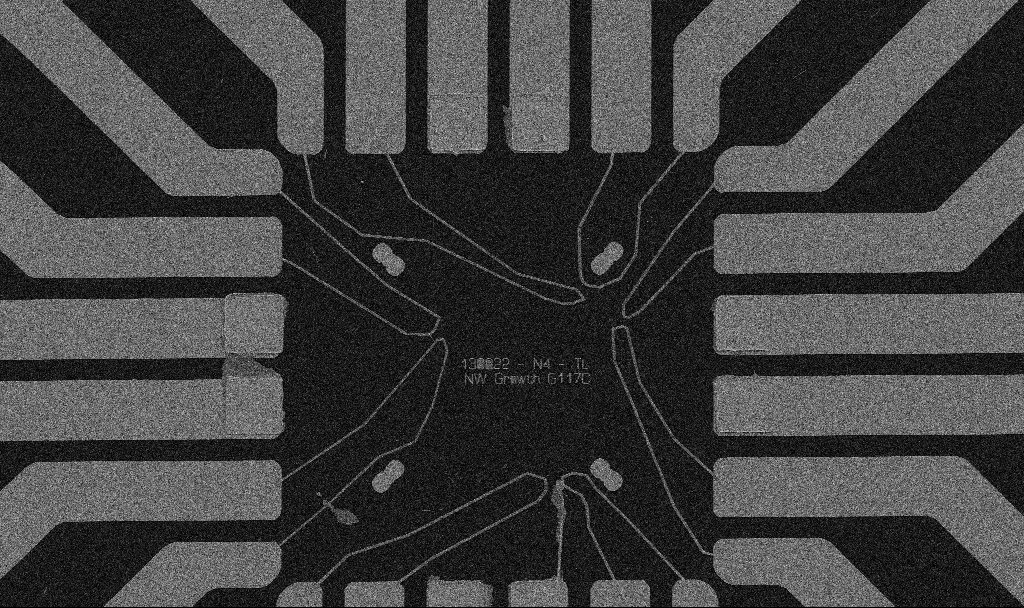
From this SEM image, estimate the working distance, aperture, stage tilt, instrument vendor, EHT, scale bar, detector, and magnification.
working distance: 10.7 mm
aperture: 30 µm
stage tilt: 0°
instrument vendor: Zeiss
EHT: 5 kV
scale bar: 20000 nm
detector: SE2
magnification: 1 K X